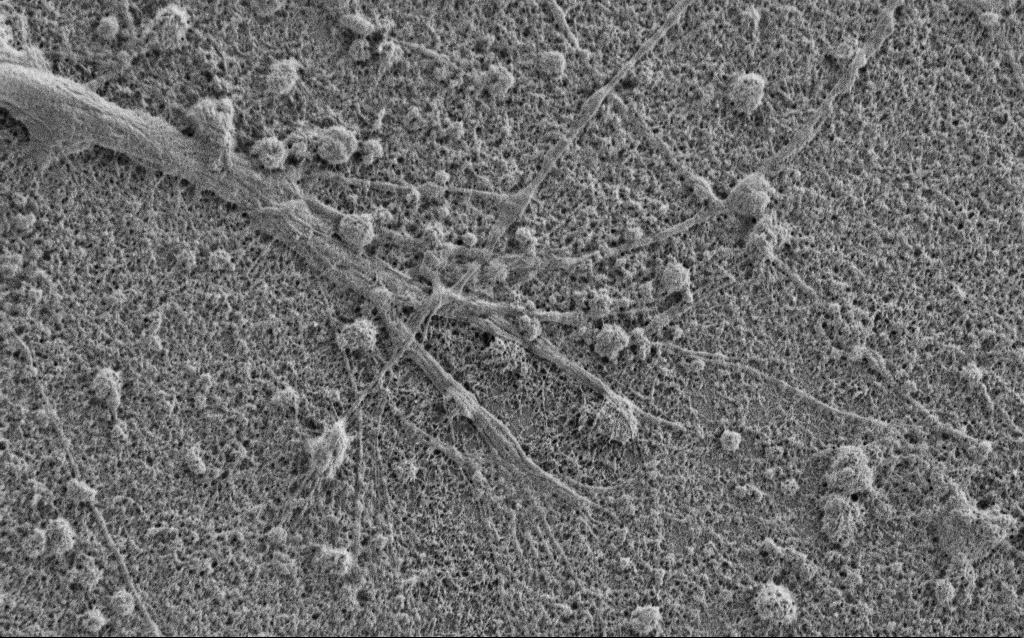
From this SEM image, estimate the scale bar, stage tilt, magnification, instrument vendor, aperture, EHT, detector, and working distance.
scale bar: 2000 nm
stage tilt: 0°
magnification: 10 K X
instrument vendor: Zeiss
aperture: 30 µm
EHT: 1 kV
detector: SE2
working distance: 5.3 mm